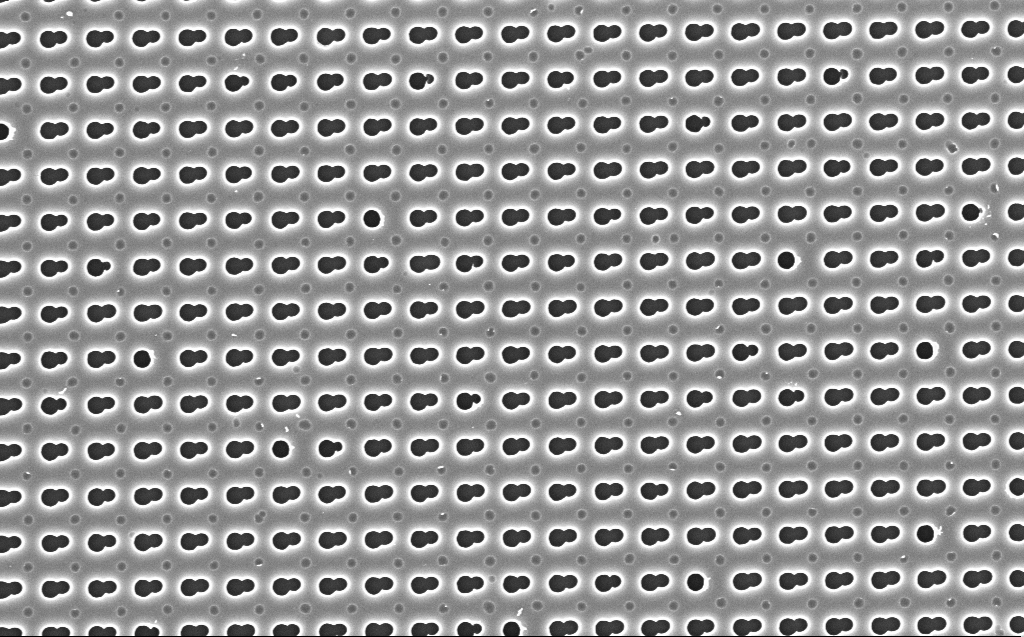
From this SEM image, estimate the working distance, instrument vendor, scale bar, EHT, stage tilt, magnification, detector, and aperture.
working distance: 4 mm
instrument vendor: Zeiss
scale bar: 2000 nm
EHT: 5 kV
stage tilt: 0°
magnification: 16.25 K X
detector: InLens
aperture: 30 µm